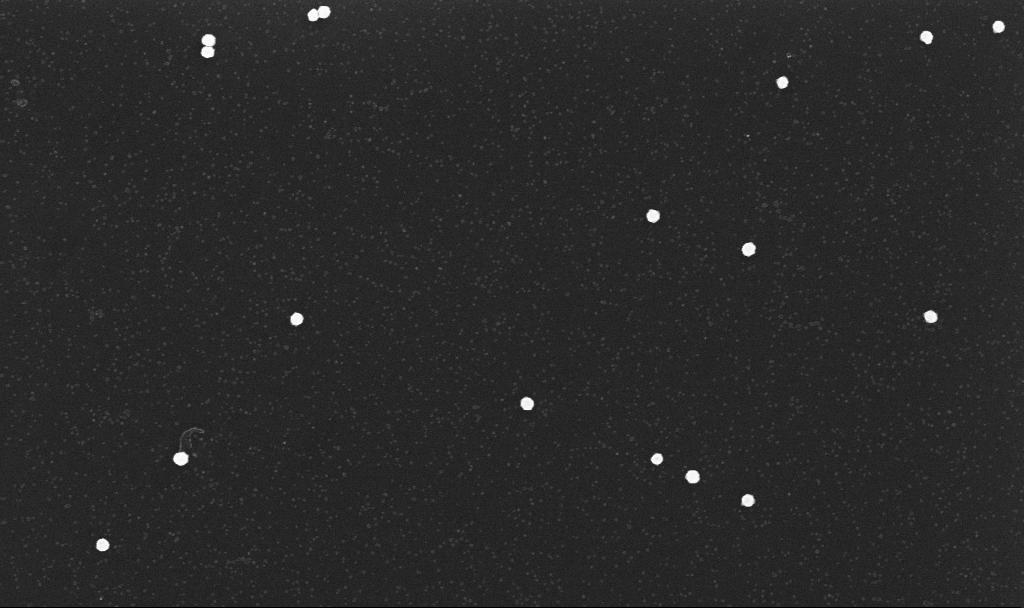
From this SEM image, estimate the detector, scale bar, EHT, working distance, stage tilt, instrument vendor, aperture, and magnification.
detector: InLens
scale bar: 1000 nm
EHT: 10 kV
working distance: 3.4 mm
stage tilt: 0°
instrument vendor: Zeiss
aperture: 30 µm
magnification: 70 K X